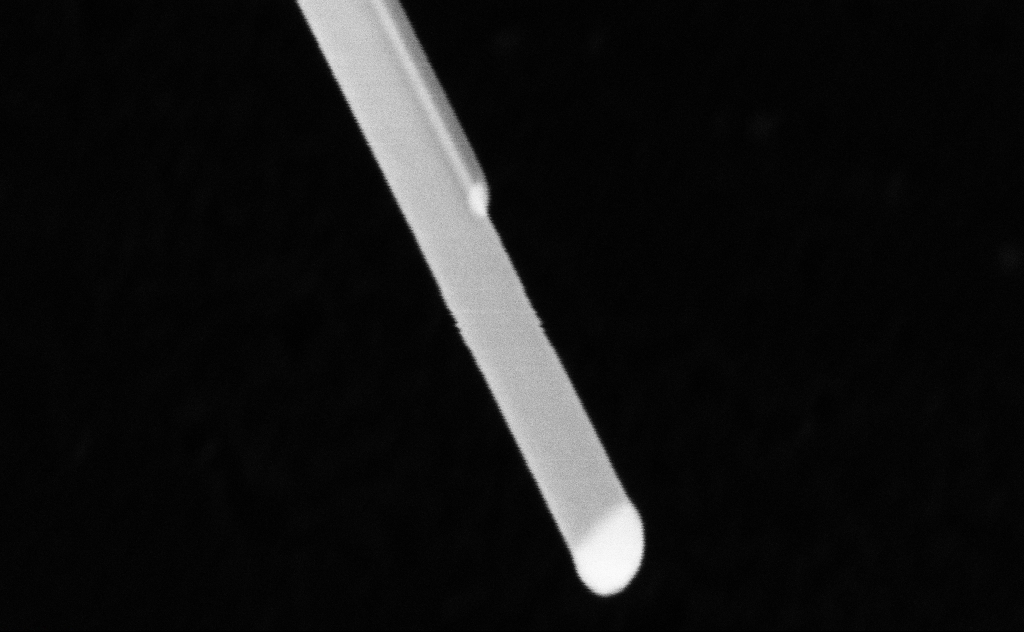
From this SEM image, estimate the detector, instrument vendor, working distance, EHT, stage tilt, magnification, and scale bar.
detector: InLens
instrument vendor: Zeiss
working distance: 8 mm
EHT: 20 kV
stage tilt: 0°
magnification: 419.36 K X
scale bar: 100 nm